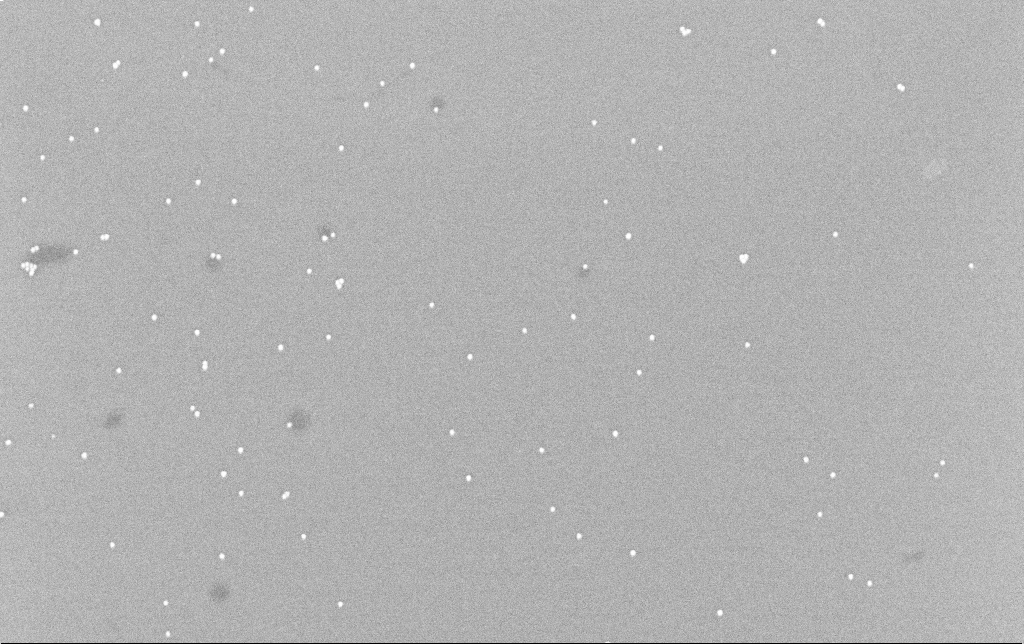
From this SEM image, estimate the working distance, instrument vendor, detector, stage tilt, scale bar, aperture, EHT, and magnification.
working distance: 6.6 mm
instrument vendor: Zeiss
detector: InLens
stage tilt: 0°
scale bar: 200 nm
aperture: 30 µm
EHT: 8 kV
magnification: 100 K X